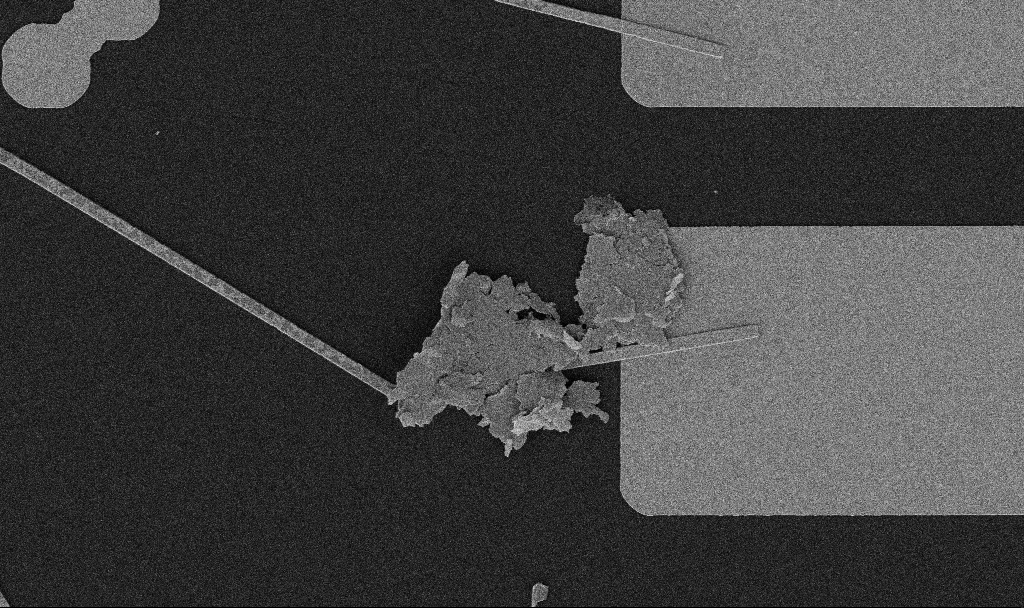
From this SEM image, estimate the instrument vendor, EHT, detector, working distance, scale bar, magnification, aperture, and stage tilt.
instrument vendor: Zeiss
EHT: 5 kV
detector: SE2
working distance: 10.7 mm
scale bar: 10000 nm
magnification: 5 K X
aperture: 30 µm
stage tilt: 0°